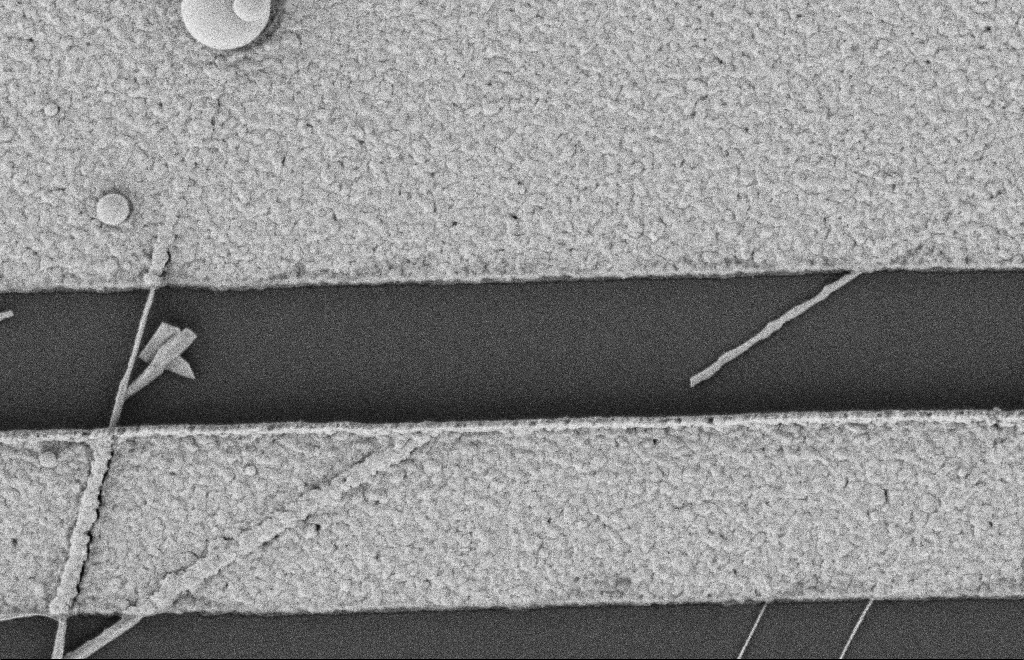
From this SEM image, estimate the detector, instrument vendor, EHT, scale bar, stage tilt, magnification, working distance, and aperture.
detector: SE2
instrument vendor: Zeiss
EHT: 2 kV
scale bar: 1000 nm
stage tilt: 0°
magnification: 29.33 K X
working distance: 12 mm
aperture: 20 µm